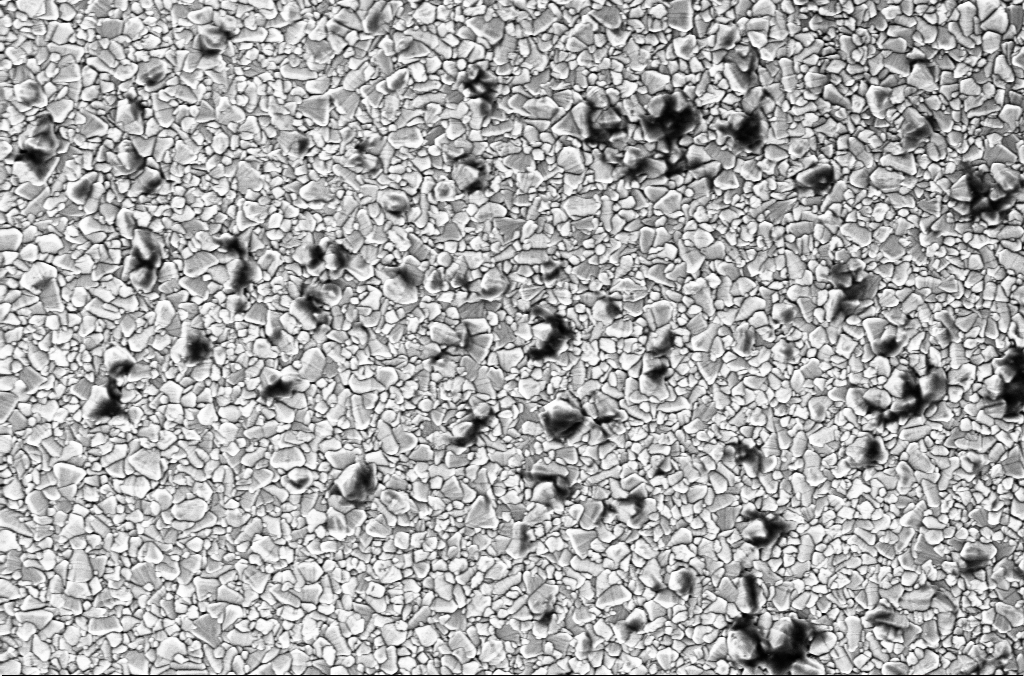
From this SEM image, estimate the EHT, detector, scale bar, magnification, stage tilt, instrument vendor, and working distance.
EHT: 2 kV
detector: InLens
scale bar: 2000 nm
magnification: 30 K X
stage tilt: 0°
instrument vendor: Zeiss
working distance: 1.9 mm